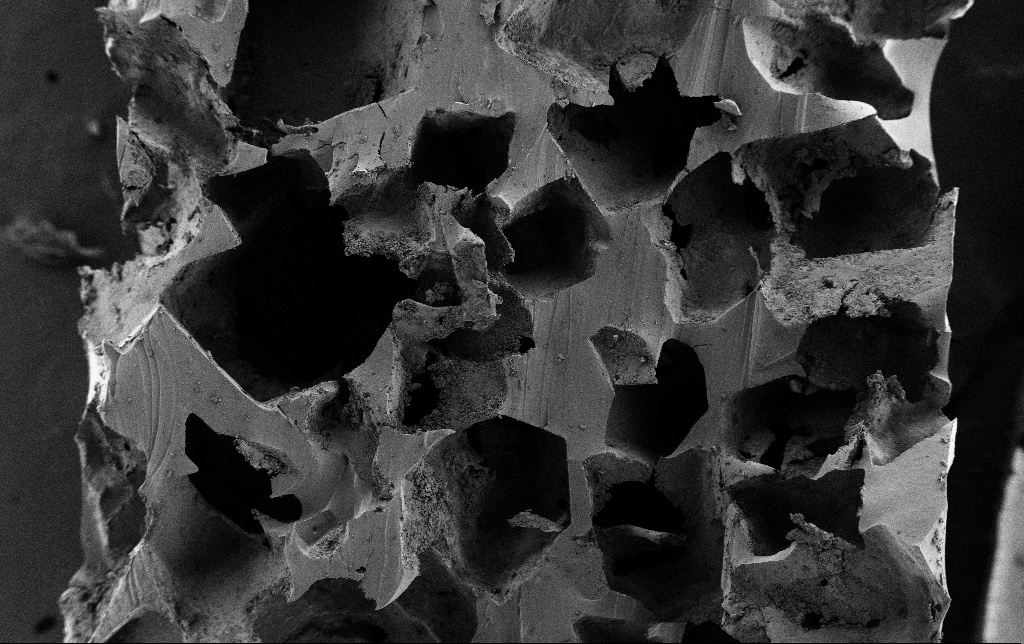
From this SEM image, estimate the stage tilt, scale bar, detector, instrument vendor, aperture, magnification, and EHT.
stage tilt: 0°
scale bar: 100000 nm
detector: SE2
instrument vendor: Zeiss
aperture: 30 µm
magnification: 0.15 K X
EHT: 3 kV